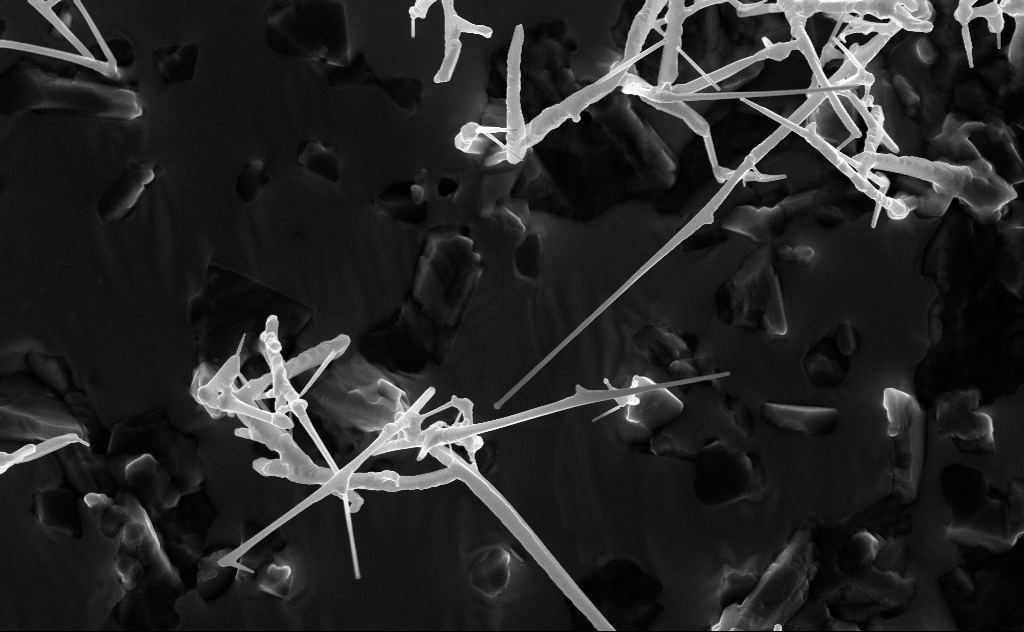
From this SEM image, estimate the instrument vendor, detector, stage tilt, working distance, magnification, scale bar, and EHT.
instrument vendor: Zeiss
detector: InLens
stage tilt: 0°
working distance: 6 mm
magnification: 20 K X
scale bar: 1000 nm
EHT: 10 kV